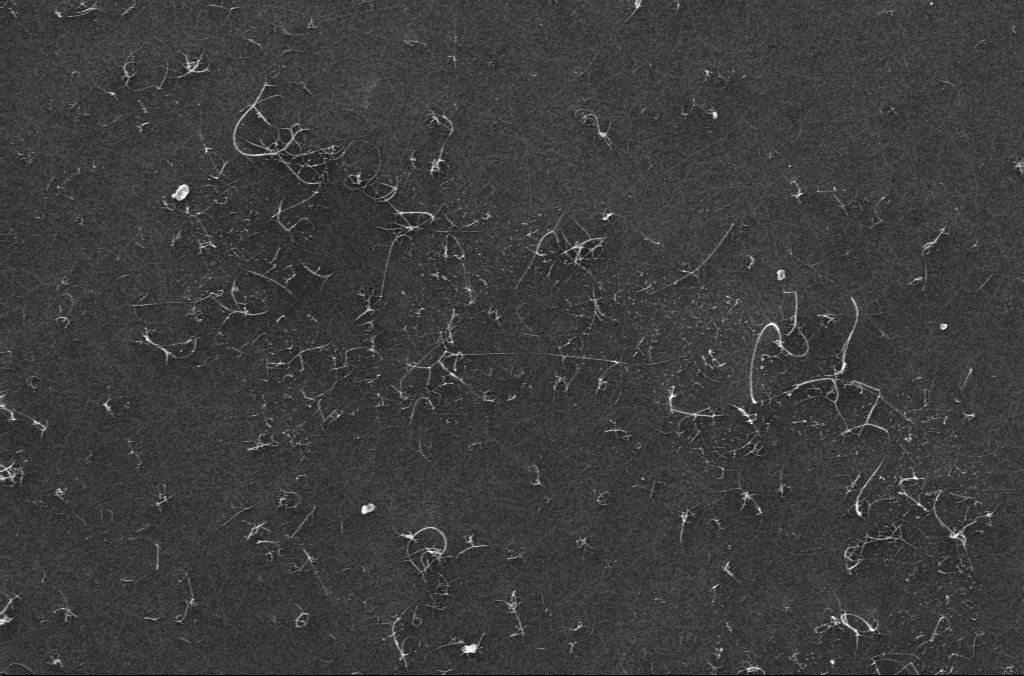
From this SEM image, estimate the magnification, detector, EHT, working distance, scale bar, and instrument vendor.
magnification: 63.08 K X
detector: InLens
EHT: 10 kV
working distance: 3.2 mm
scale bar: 1000 nm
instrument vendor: Zeiss